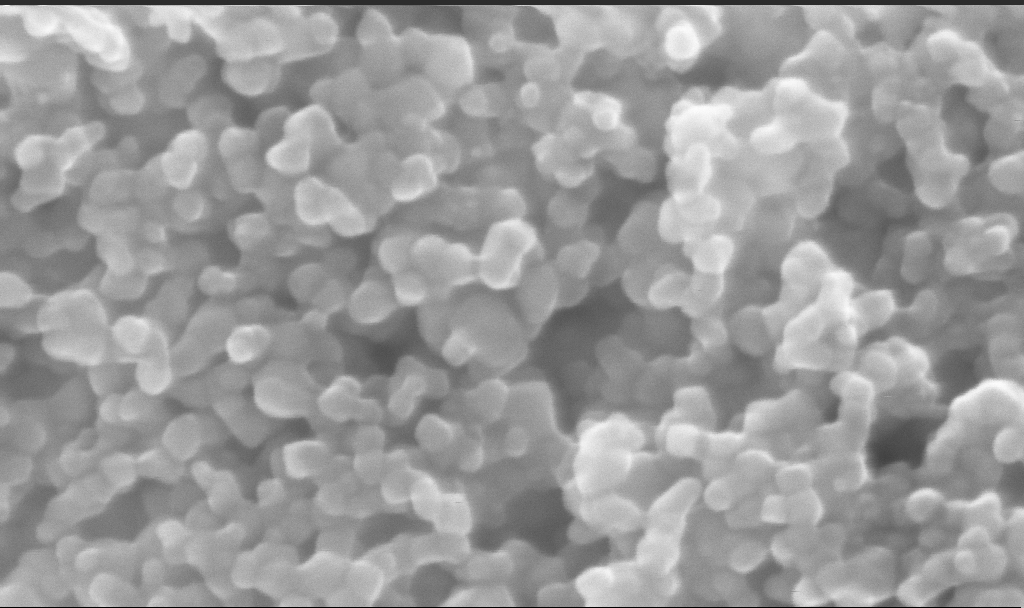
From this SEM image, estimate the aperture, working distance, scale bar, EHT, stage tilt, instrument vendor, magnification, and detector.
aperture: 30 µm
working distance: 2.7 mm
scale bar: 100 nm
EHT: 10 kV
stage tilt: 0°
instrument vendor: Zeiss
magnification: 453.83 K X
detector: InLens